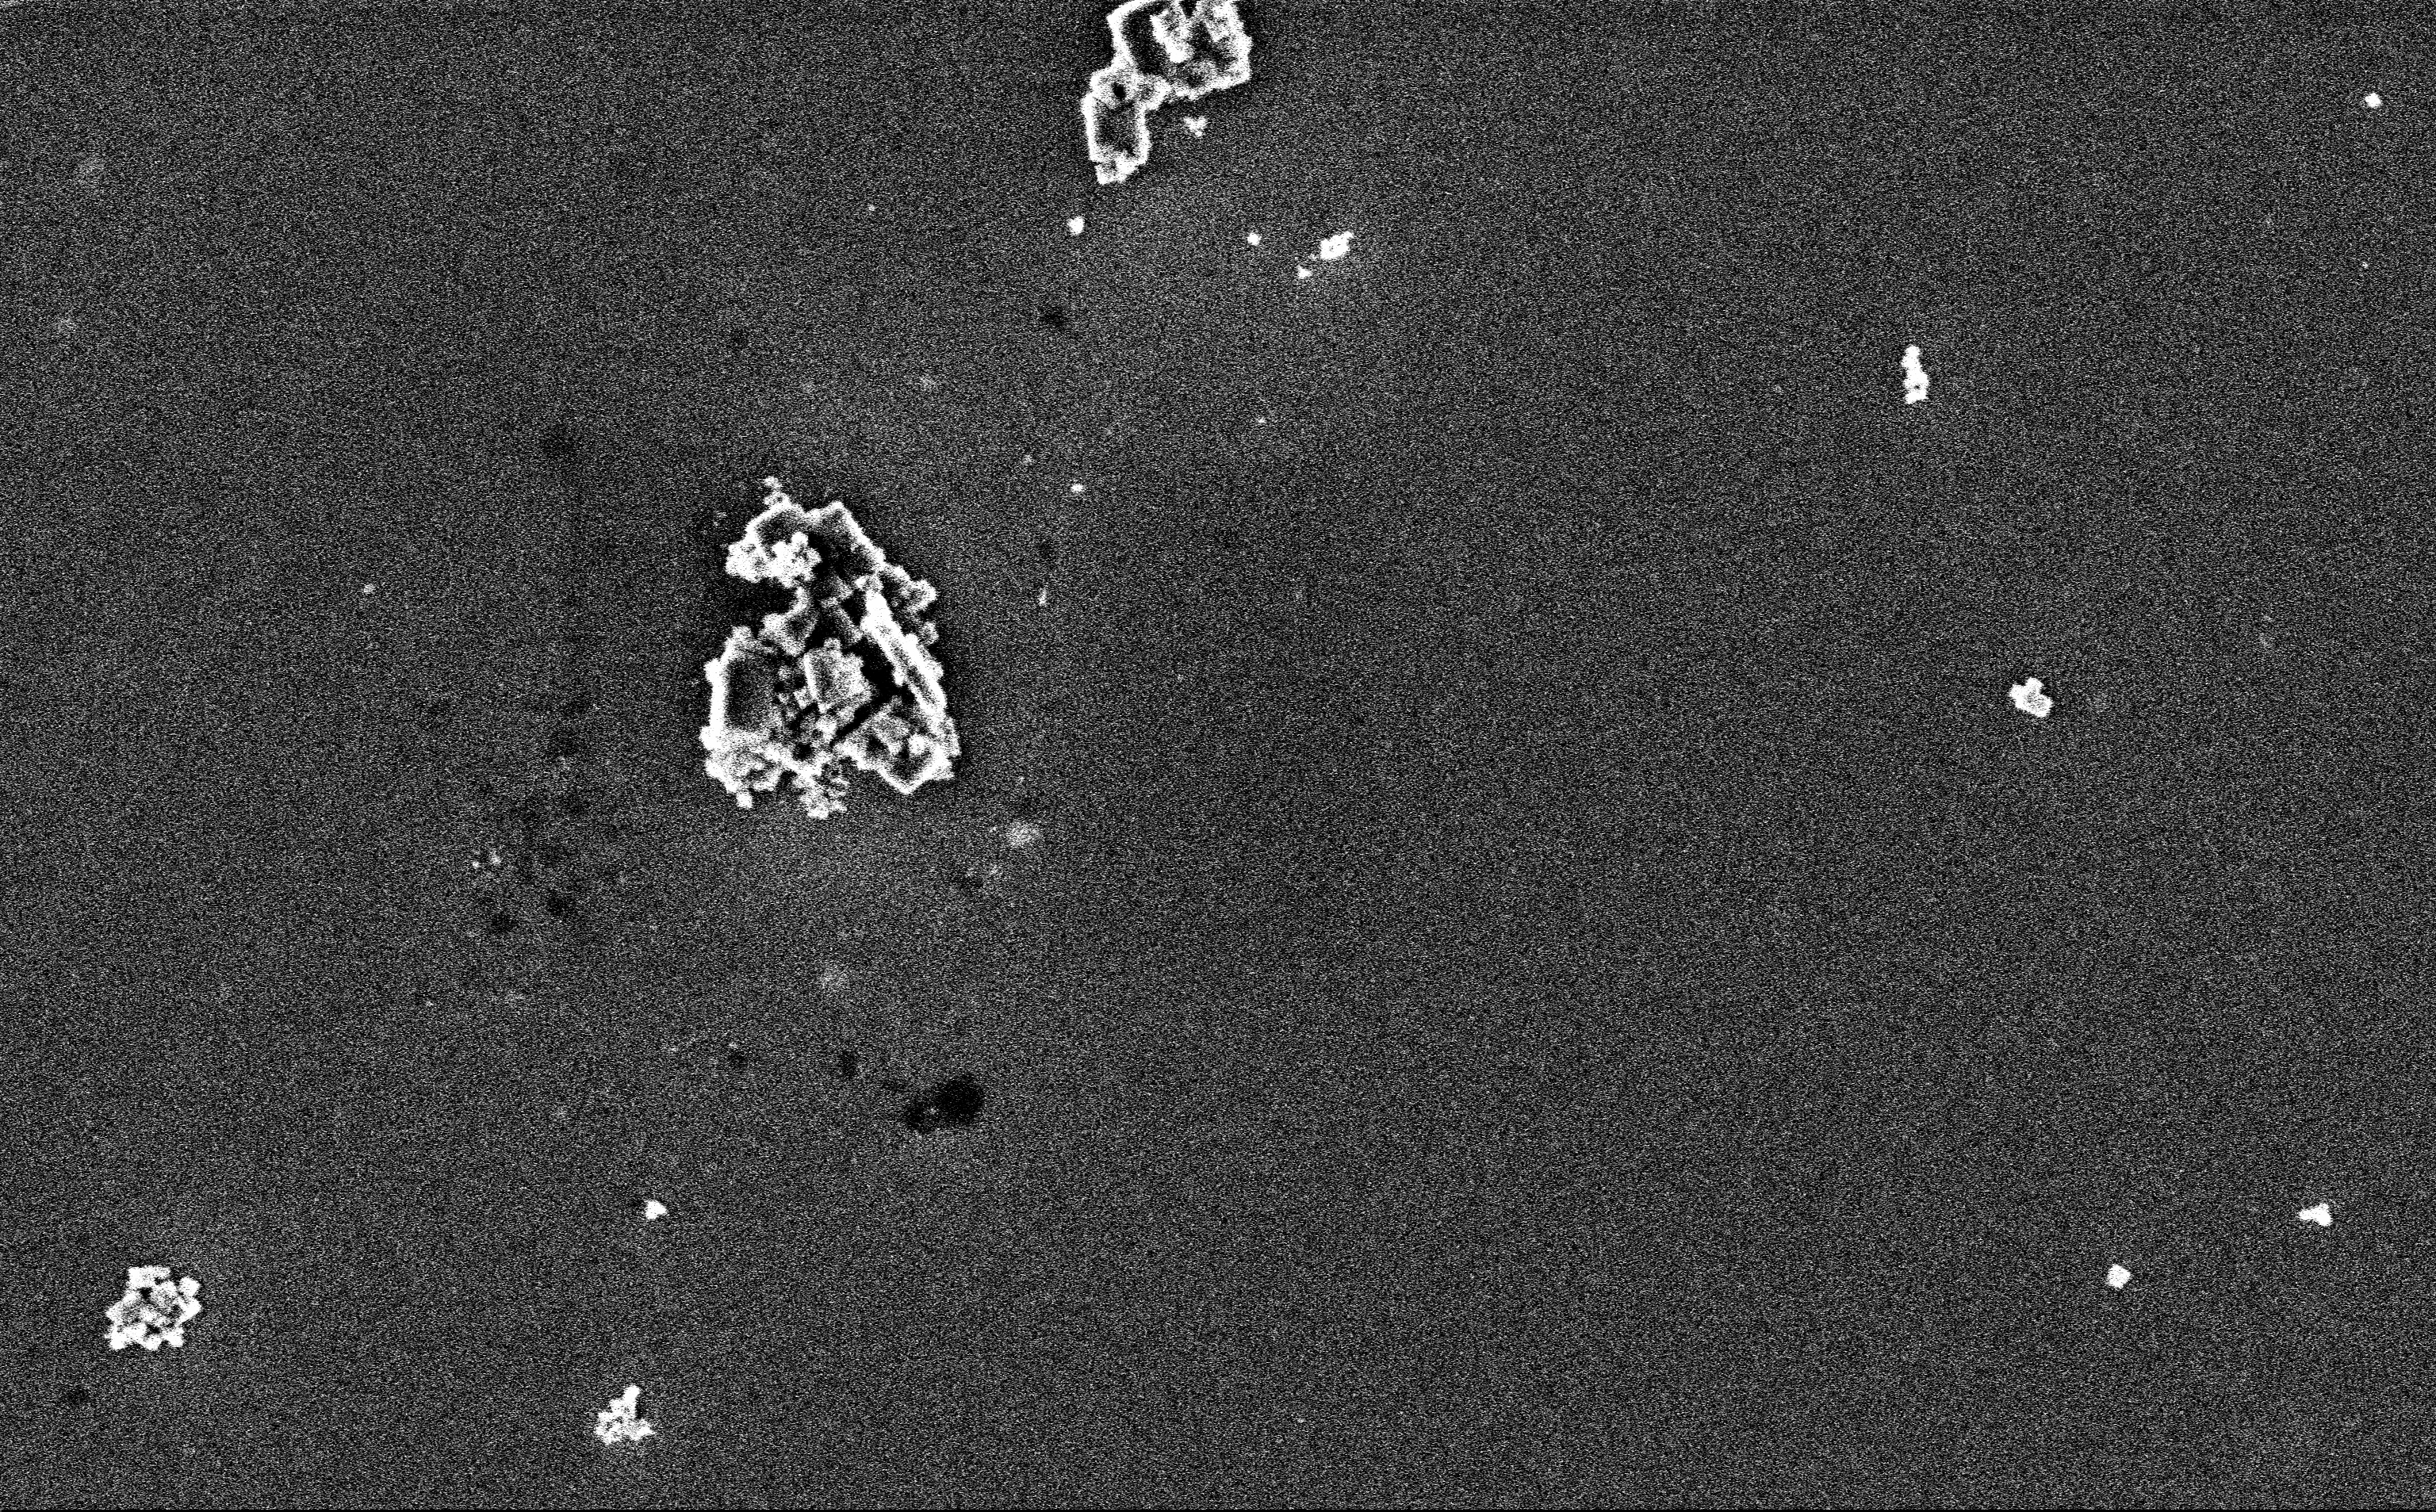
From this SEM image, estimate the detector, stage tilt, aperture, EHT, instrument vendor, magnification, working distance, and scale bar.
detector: InLens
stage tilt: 0°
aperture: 30 µm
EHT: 3 kV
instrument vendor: Zeiss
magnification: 19.01 K X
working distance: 3 mm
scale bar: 2000 nm